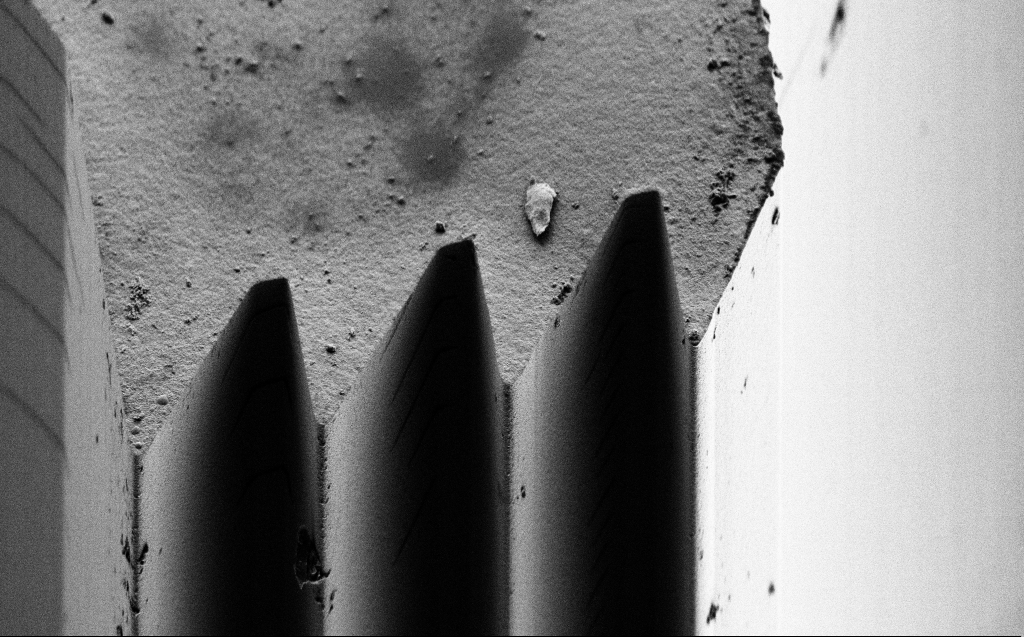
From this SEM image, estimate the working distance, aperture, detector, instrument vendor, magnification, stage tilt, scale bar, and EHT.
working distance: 6 mm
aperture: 30 µm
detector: SE2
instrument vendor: Zeiss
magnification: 6.08 K X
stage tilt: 45°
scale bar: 10000 nm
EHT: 2 kV